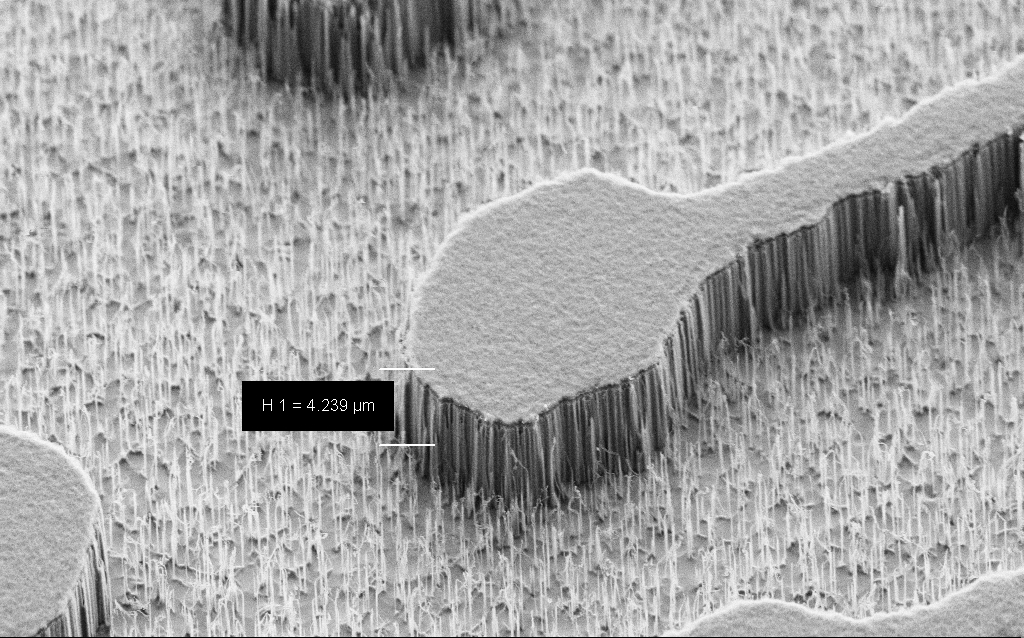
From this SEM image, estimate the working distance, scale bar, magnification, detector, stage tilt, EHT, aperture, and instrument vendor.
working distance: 7 mm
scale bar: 10000 nm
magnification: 6.58 K X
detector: SE2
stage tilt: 45°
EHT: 3 kV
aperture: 30 µm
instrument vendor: Zeiss